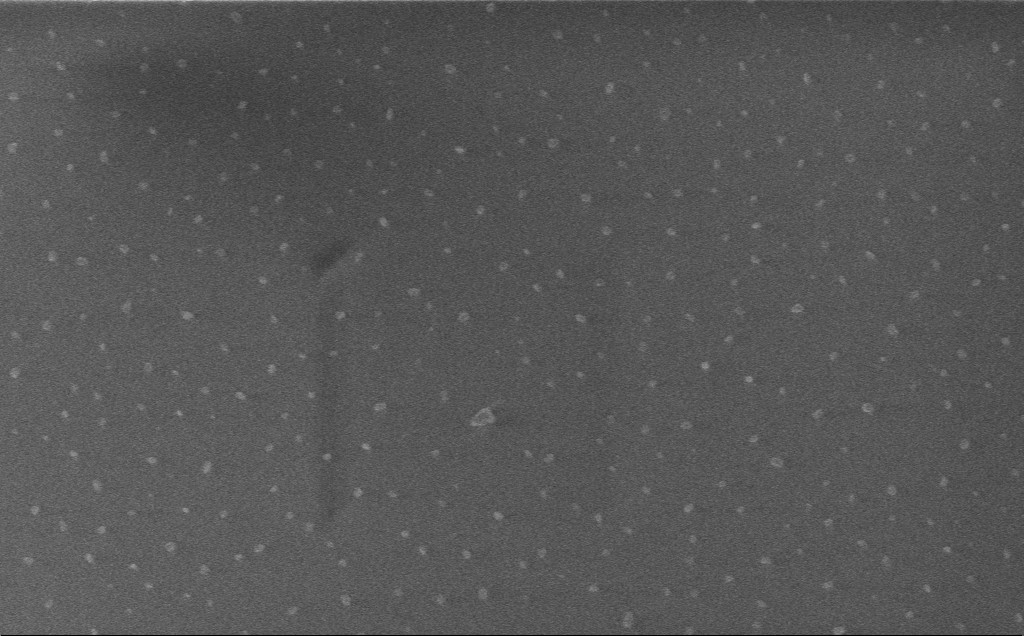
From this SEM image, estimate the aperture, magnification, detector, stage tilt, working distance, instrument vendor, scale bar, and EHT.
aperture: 30 µm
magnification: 42.26 K X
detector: InLens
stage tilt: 0°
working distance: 3 mm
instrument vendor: Zeiss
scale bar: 1000 nm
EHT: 1 kV